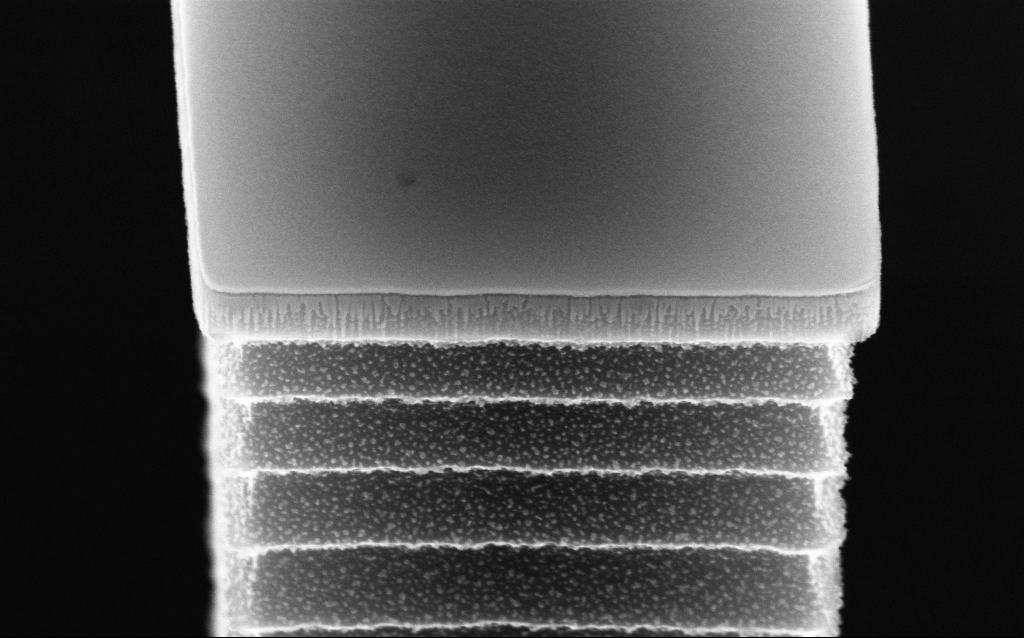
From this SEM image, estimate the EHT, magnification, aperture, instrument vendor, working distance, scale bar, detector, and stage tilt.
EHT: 10 kV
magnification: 125.43 K X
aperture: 30 µm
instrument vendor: Zeiss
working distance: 4.8 mm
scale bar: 200 nm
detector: InLens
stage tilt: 45°